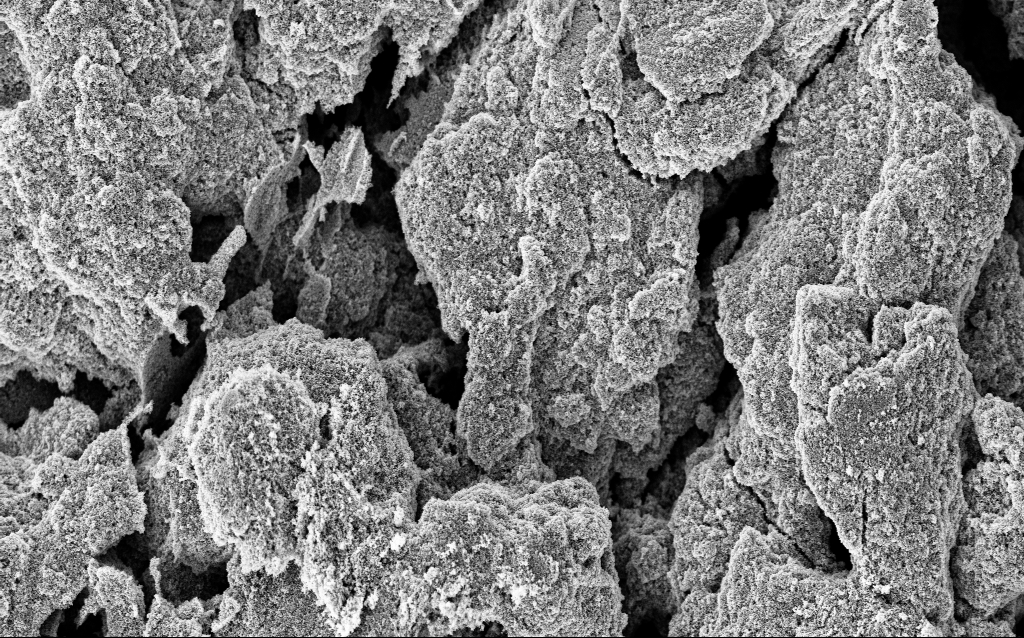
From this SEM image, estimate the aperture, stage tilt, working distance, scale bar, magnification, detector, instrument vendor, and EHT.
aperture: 30 µm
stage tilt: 0°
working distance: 9.6 mm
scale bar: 10000 nm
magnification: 5.4 K X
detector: InLens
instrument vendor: Zeiss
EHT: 5 kV